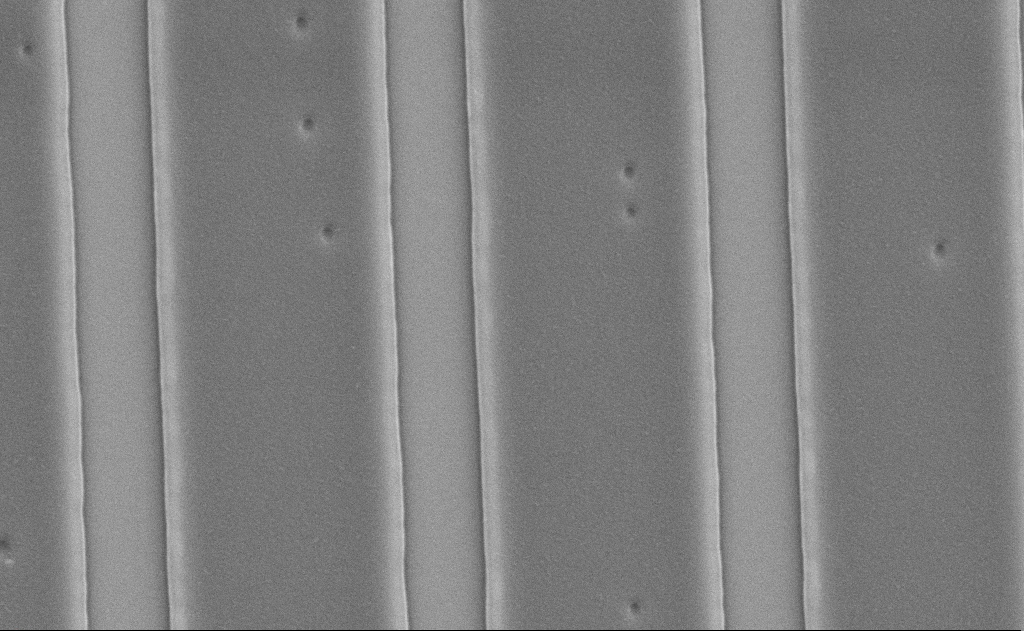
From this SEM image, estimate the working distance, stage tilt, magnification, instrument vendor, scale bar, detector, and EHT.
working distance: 12 mm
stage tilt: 0°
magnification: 28.98 K X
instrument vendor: Zeiss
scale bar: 2000 nm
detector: SE2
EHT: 5 kV